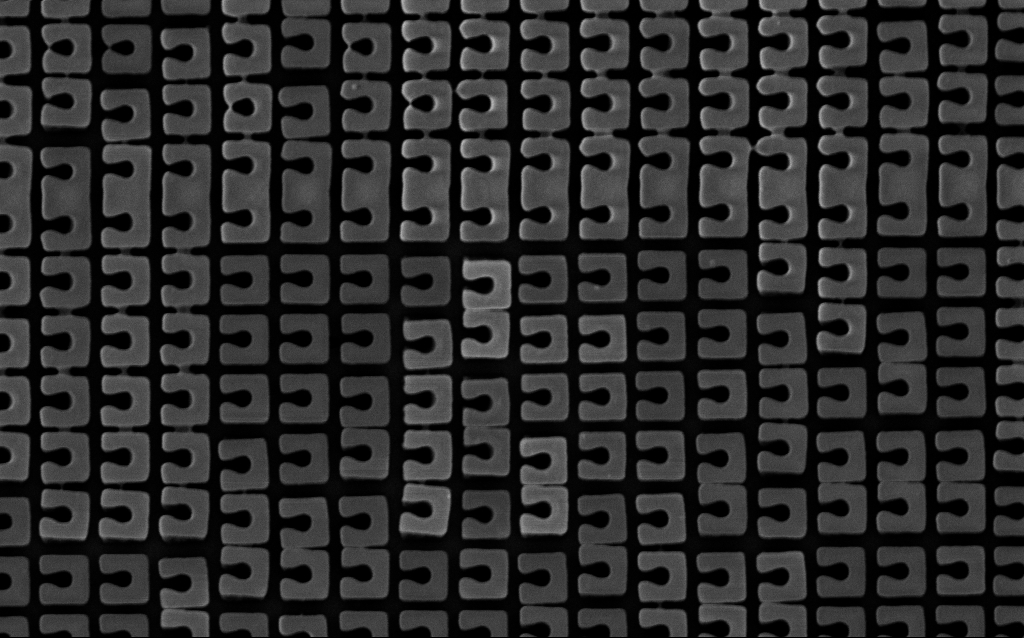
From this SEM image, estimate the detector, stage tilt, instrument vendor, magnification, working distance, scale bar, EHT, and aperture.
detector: InLens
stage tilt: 0°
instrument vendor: Zeiss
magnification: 47.71 K X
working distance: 4.3 mm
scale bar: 1000 nm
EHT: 3 kV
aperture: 30 µm